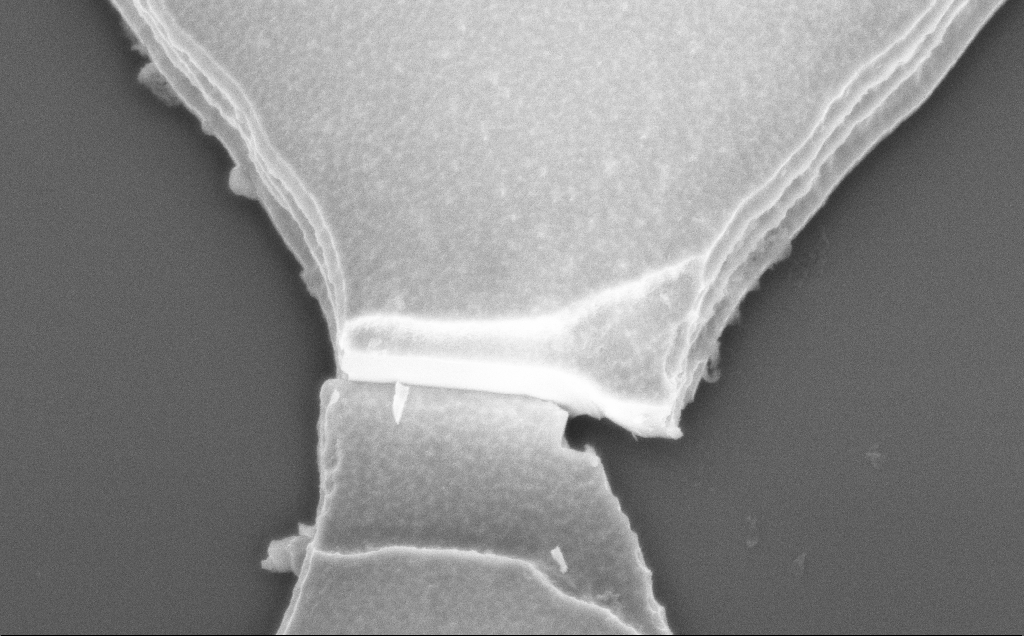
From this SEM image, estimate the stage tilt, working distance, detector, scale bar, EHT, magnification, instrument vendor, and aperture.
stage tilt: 22.9°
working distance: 8 mm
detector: InLens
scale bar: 1000 nm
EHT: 4 kV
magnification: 25.46 K X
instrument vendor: Zeiss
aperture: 30 µm